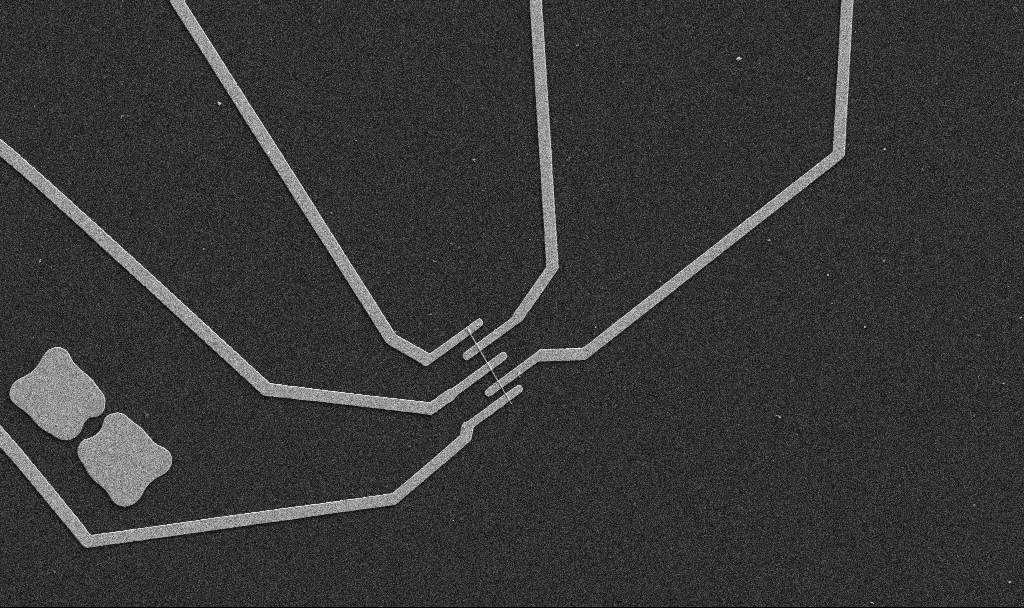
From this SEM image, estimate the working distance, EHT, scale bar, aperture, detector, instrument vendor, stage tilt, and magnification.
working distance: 10.7 mm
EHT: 5 kV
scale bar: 10000 nm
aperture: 30 µm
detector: SE2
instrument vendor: Zeiss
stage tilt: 0°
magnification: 5 K X